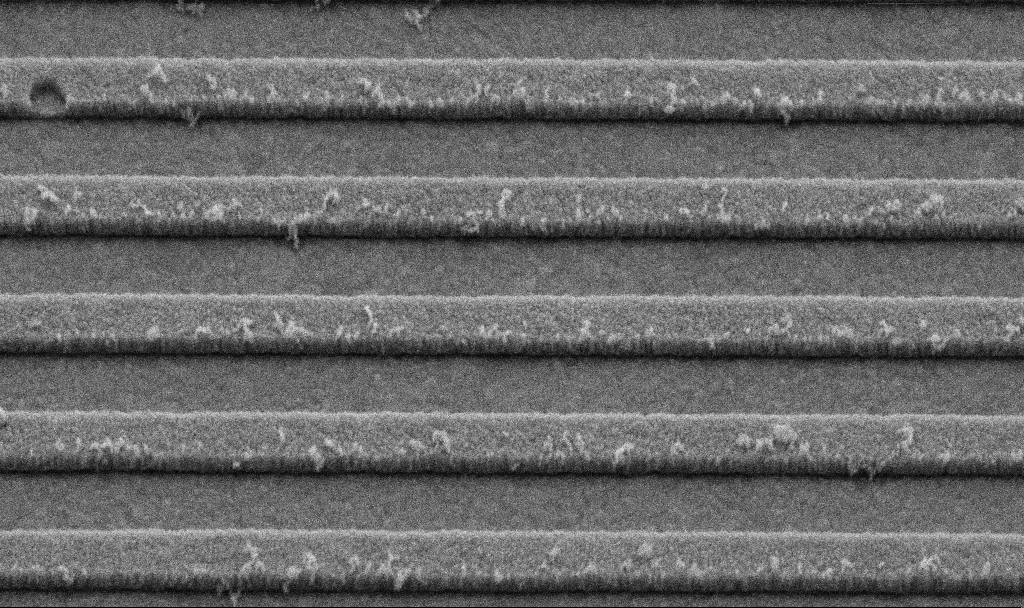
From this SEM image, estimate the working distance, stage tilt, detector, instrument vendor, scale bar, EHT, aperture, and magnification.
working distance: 8 mm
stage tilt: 45°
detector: SE2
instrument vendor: Zeiss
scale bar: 200 nm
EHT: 5 kV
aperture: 30 µm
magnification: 73.72 K X